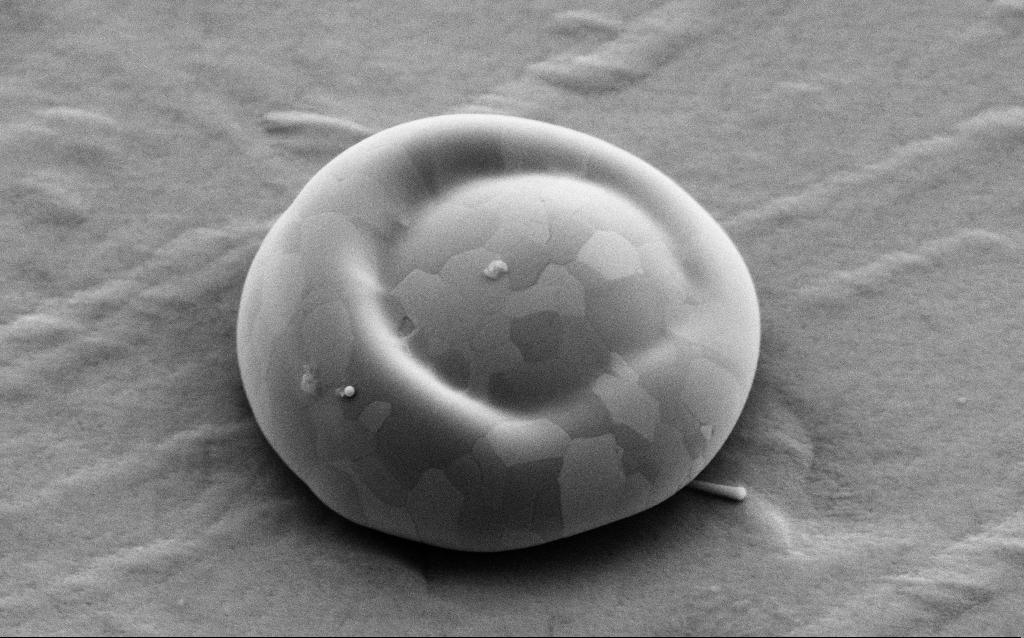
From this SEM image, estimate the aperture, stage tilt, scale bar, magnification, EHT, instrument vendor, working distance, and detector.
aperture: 30 µm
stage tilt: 35°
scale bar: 1000 nm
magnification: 42.81 K X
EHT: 5 kV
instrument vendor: Zeiss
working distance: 4 mm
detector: SE2